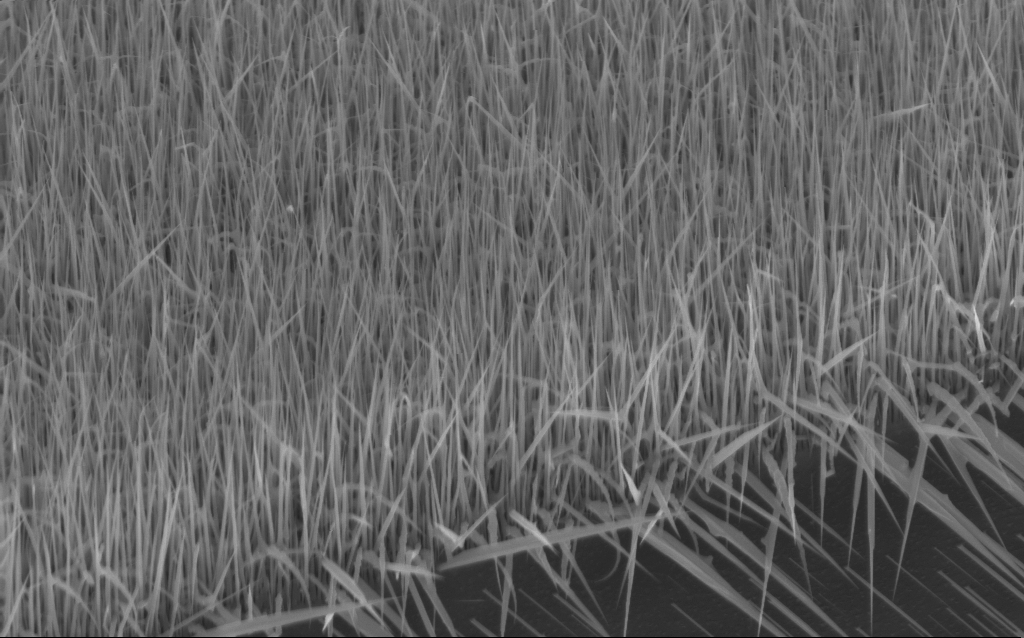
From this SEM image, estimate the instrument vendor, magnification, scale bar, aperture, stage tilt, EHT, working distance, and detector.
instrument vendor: Zeiss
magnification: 26.8 K X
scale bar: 1000 nm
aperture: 30 µm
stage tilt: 45°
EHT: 10 kV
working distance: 6 mm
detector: InLens